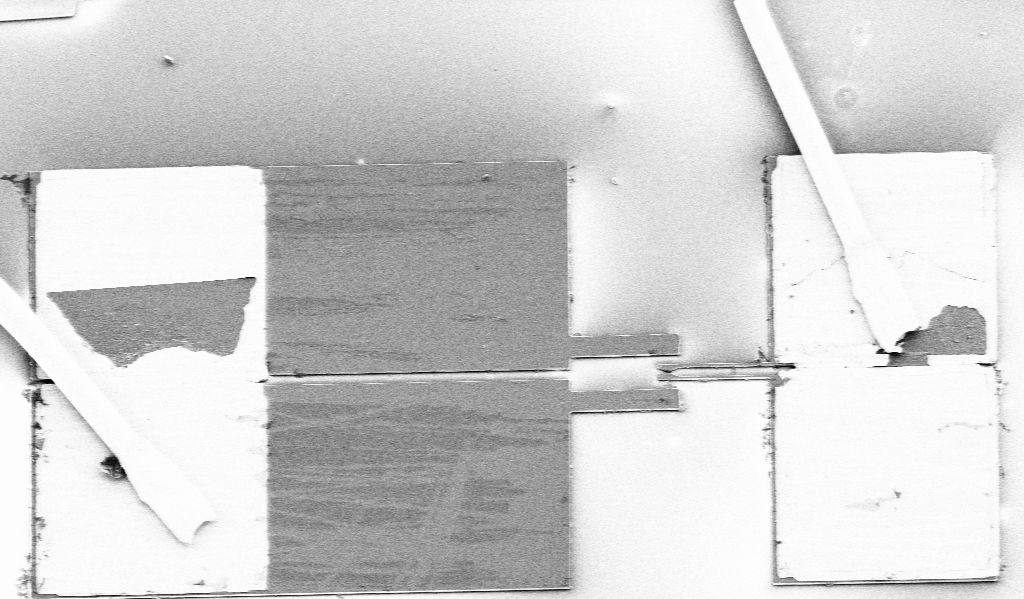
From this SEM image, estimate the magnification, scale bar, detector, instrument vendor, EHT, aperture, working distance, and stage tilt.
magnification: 0.413 K X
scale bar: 100000 nm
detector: SE2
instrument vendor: Zeiss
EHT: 5 kV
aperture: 30 µm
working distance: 13 mm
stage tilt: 24.6°